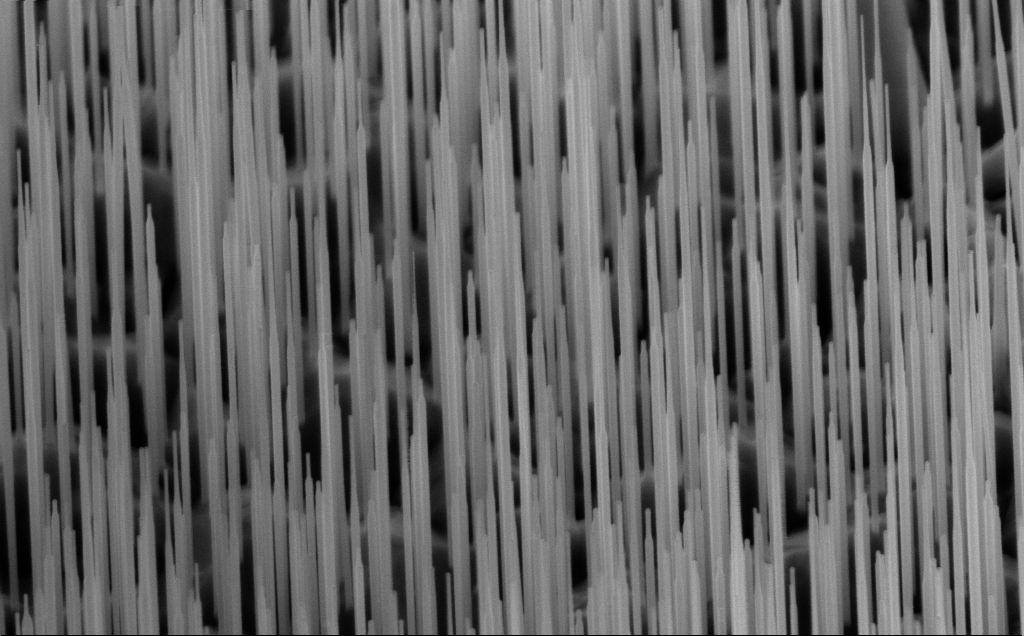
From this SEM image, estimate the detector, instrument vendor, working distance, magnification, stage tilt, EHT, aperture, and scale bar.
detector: InLens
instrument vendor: Zeiss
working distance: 6 mm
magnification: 40 K X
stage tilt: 45°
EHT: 10 kV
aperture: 30 µm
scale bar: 1000 nm